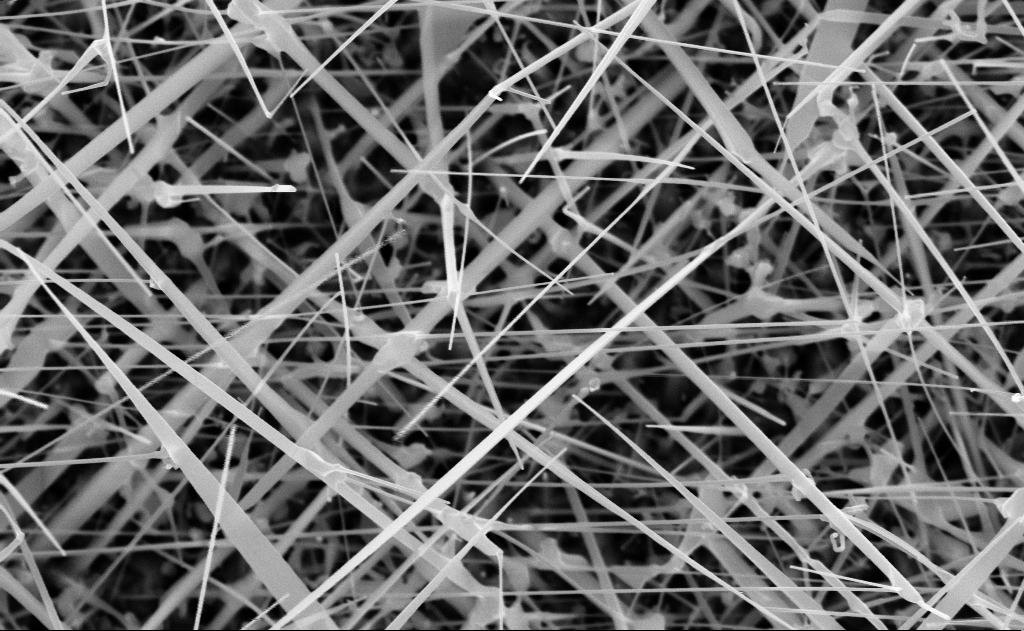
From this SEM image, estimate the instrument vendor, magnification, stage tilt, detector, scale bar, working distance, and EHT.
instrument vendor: Zeiss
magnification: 40 K X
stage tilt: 0°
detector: InLens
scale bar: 1000 nm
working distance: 11 mm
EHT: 10 kV